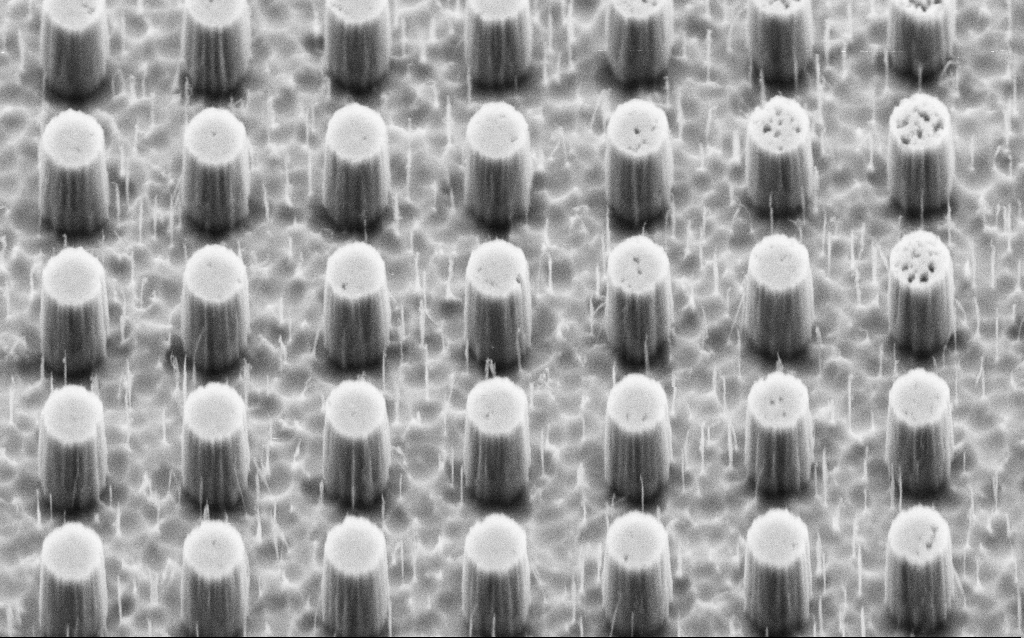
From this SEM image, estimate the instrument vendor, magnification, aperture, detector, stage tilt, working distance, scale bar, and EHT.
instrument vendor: Zeiss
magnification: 43.67 K X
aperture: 30 µm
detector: SE2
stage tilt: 45°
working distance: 5 mm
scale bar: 1000 nm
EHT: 3 kV